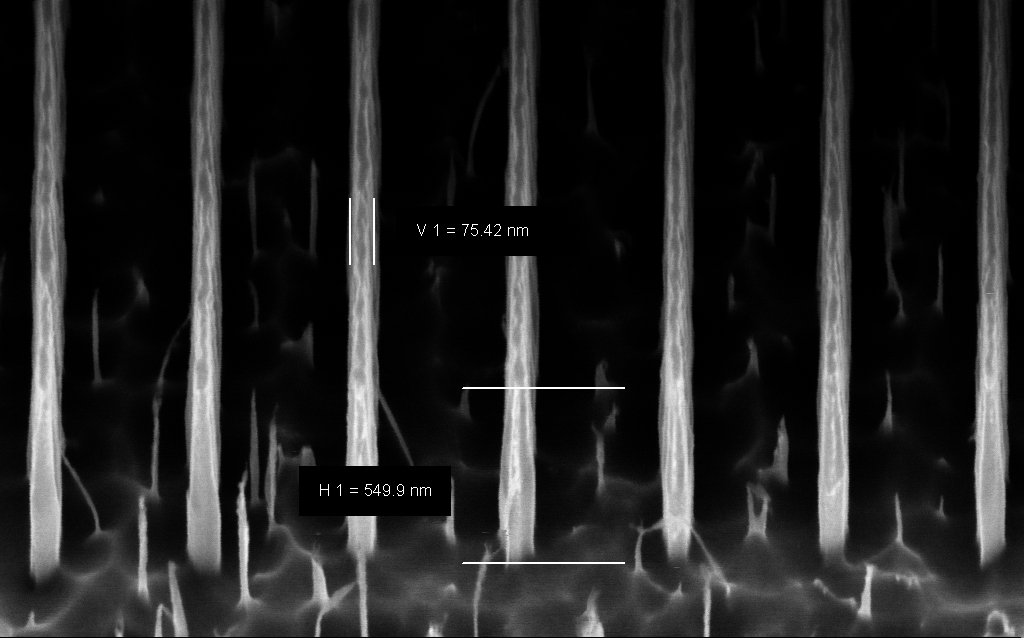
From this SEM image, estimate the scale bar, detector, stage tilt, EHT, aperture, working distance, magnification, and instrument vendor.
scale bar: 200 nm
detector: InLens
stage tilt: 45°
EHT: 3 kV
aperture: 30 µm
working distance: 5 mm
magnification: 116.85 K X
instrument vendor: Zeiss